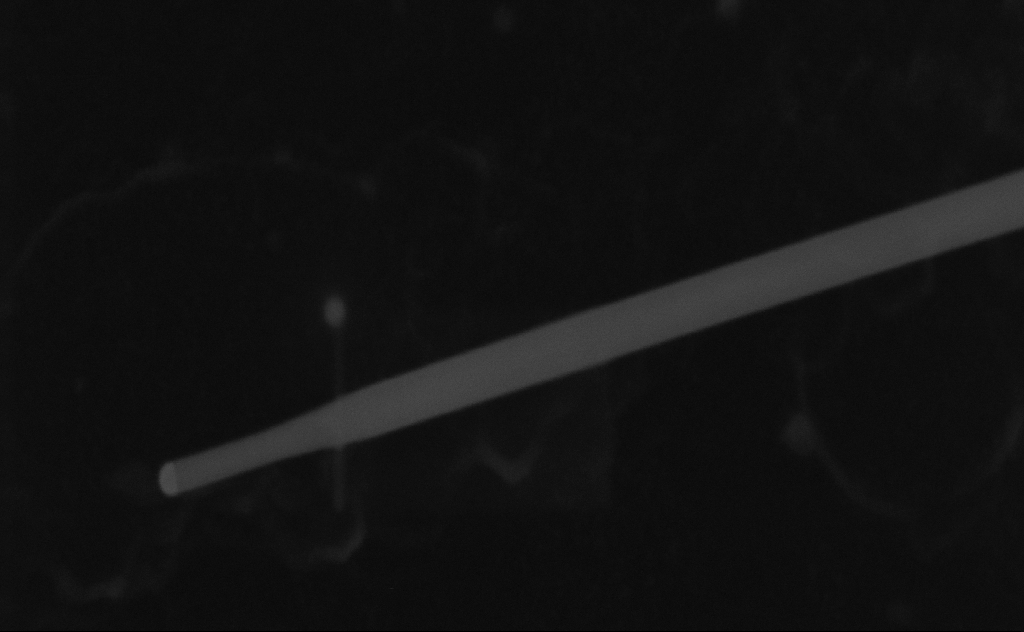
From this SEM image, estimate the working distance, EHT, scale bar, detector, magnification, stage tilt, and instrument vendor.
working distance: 8 mm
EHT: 20 kV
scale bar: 100 nm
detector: SE2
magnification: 371.83 K X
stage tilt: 0°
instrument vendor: Zeiss